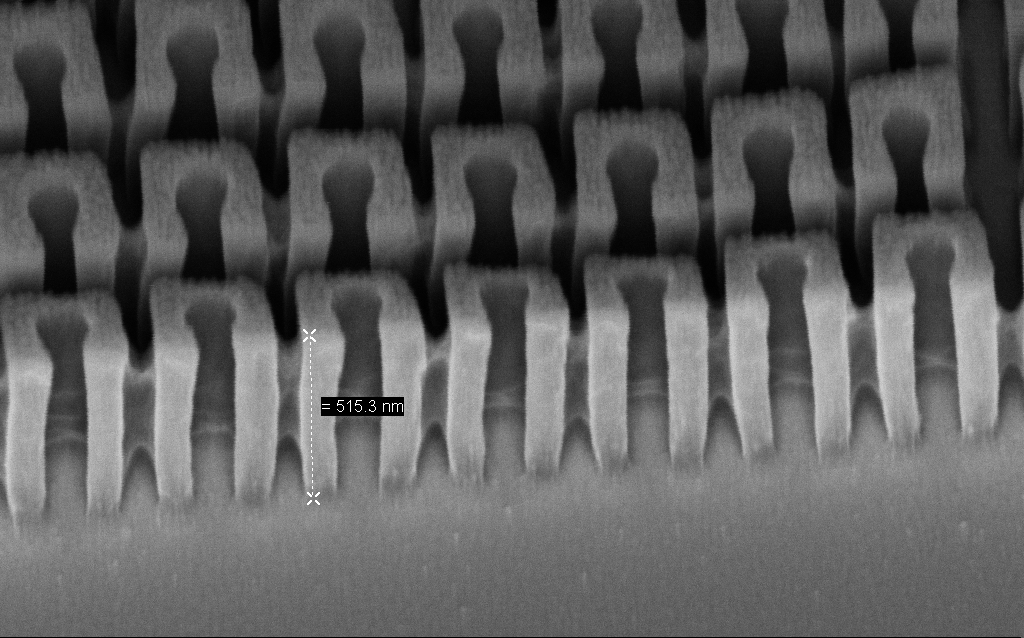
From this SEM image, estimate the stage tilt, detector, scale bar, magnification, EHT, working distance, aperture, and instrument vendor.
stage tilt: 45°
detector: InLens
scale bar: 200 nm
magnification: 116.19 K X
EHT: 3 kV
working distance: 7.3 mm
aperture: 30 µm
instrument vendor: Zeiss